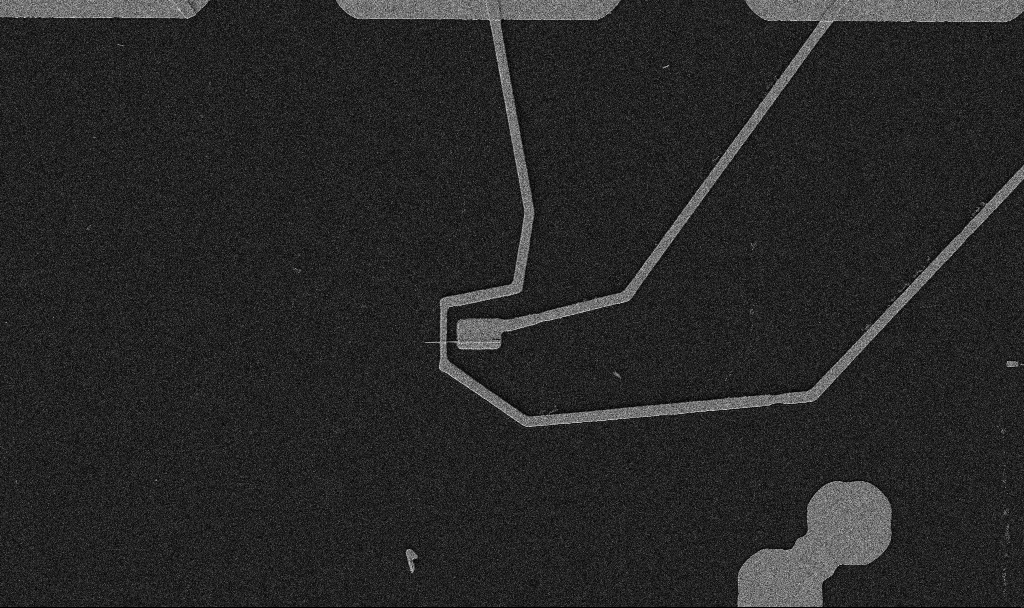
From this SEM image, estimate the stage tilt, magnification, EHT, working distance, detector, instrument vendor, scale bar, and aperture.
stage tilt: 0°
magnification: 5 K X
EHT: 5 kV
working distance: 10.7 mm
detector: SE2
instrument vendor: Zeiss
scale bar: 10000 nm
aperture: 30 µm